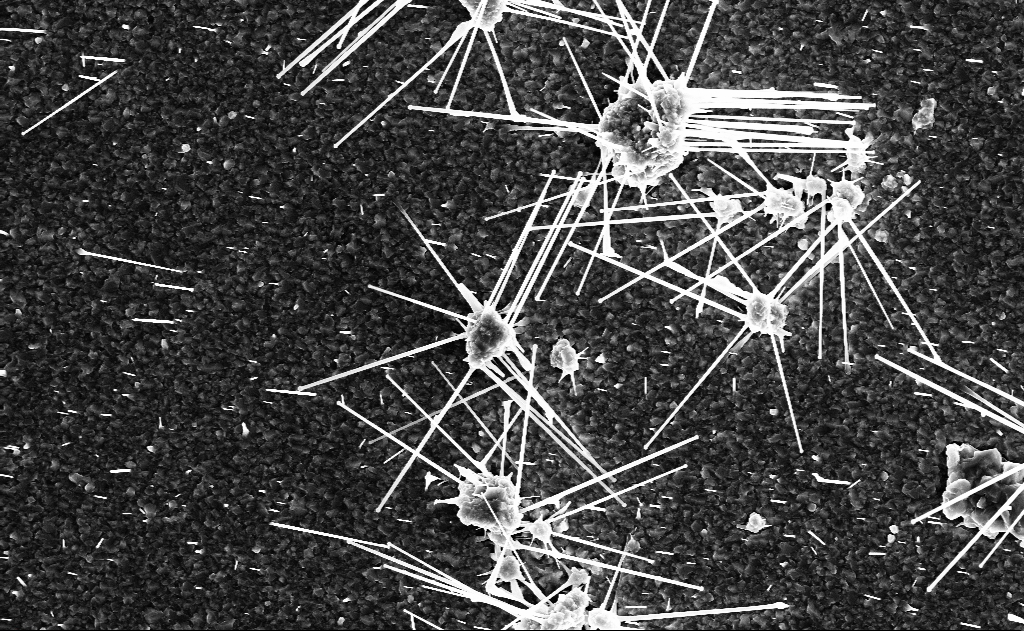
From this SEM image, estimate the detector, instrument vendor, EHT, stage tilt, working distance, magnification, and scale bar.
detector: InLens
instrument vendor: Zeiss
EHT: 10 kV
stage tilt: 0°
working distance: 9 mm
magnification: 10 K X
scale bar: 2000 nm